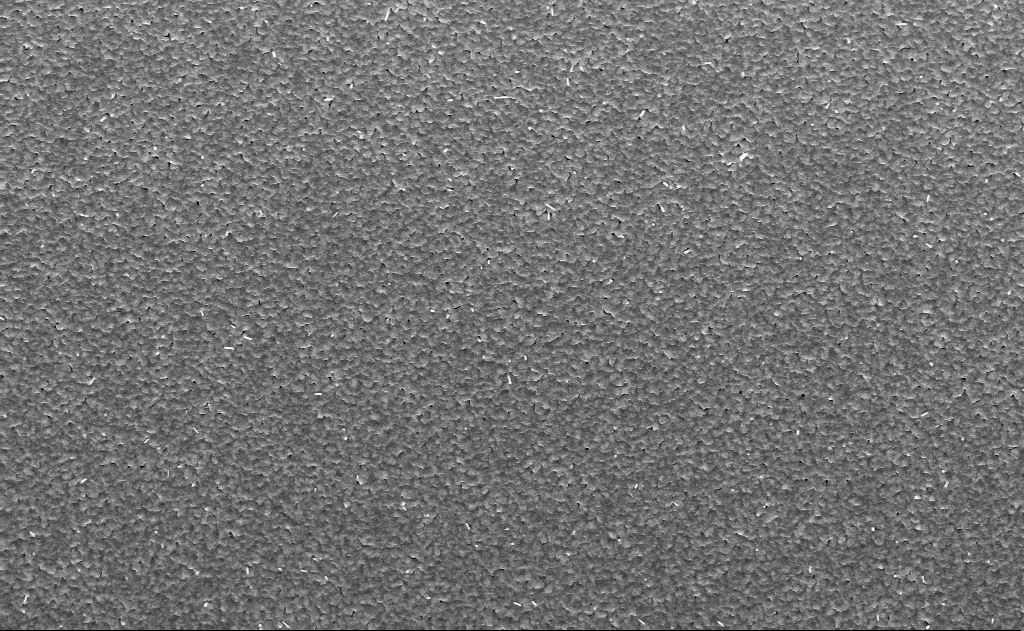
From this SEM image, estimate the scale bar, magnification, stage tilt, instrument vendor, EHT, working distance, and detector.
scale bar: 2000 nm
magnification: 10 K X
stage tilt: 0°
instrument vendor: Zeiss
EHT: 10 kV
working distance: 15 mm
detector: InLens